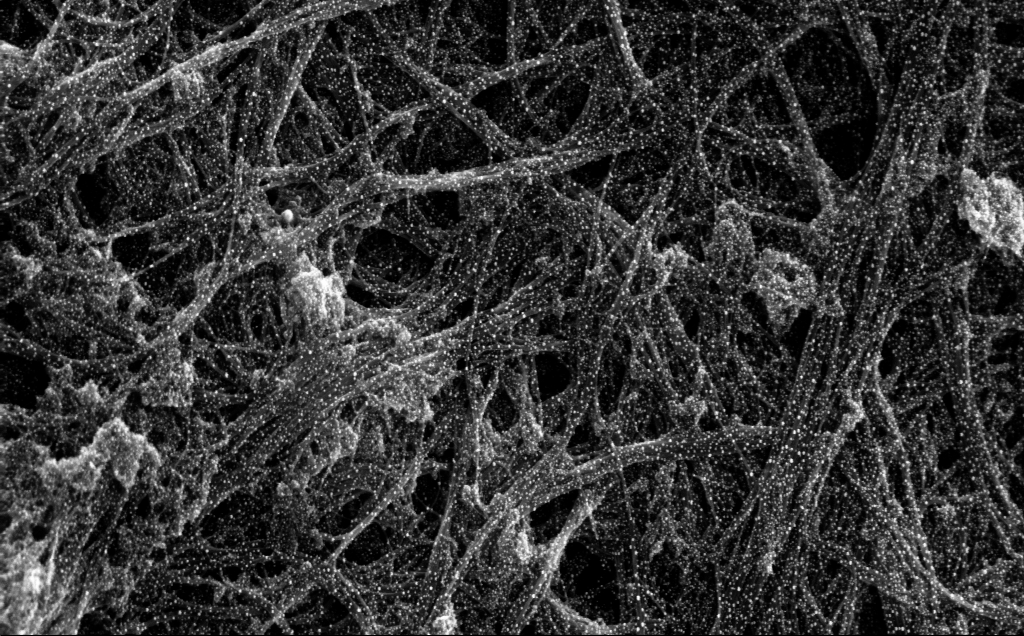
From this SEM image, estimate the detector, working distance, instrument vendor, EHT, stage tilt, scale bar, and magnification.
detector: InLens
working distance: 4 mm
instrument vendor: Zeiss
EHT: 5 kV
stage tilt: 0°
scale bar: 200 nm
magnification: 91.52 K X